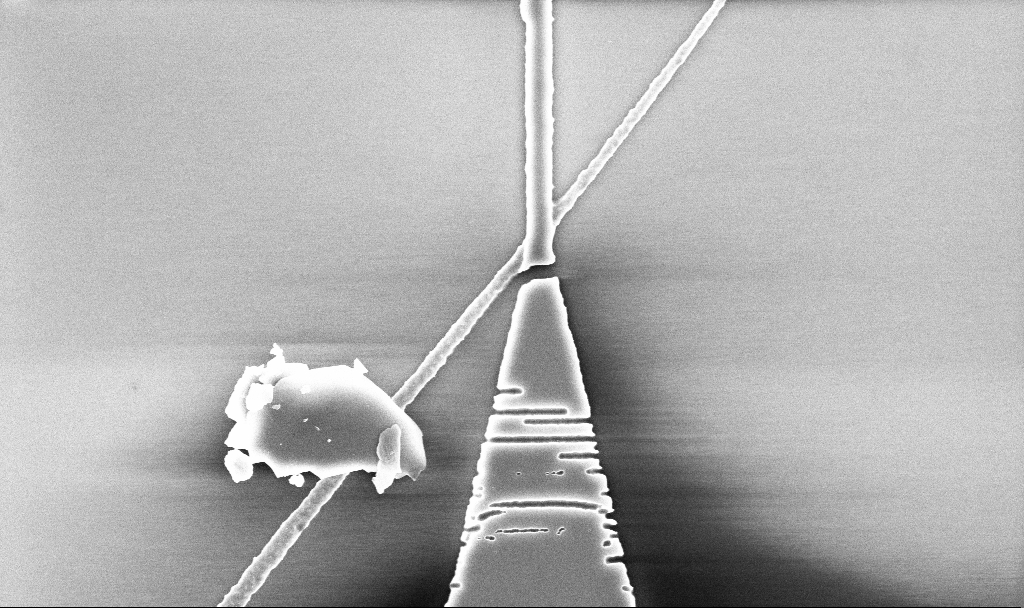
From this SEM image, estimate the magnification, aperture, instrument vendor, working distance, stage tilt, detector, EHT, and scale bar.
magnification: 18.69 K X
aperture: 30 µm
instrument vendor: Zeiss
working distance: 5.2 mm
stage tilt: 0°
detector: InLens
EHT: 5 kV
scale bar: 2000 nm